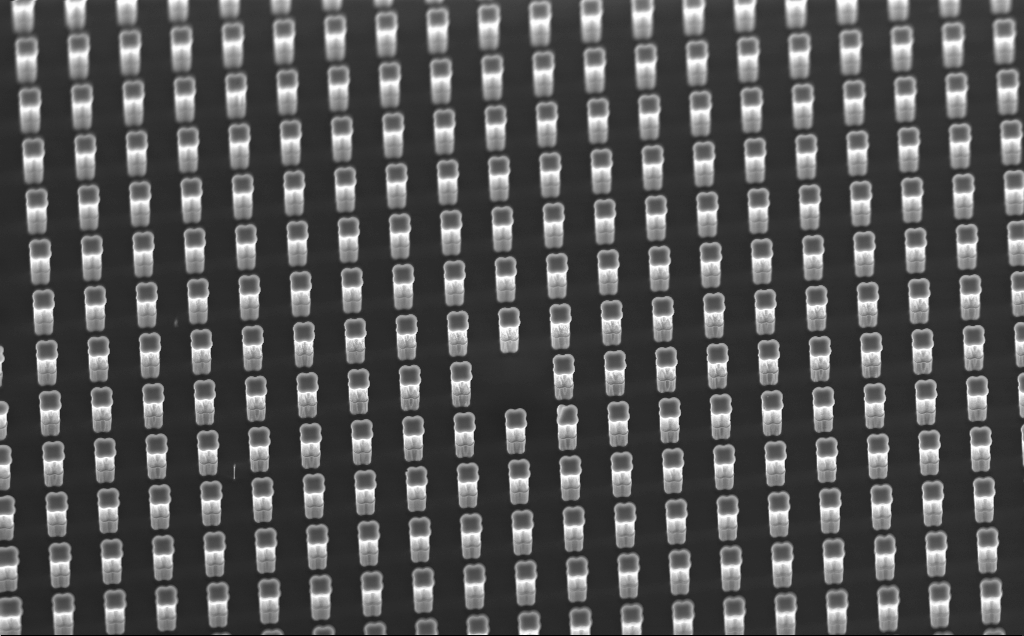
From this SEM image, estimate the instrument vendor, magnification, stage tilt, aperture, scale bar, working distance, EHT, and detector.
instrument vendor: Zeiss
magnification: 1.89 K X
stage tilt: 30.3°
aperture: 120 µm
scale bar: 20000 nm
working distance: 9 mm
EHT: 10 kV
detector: InLens